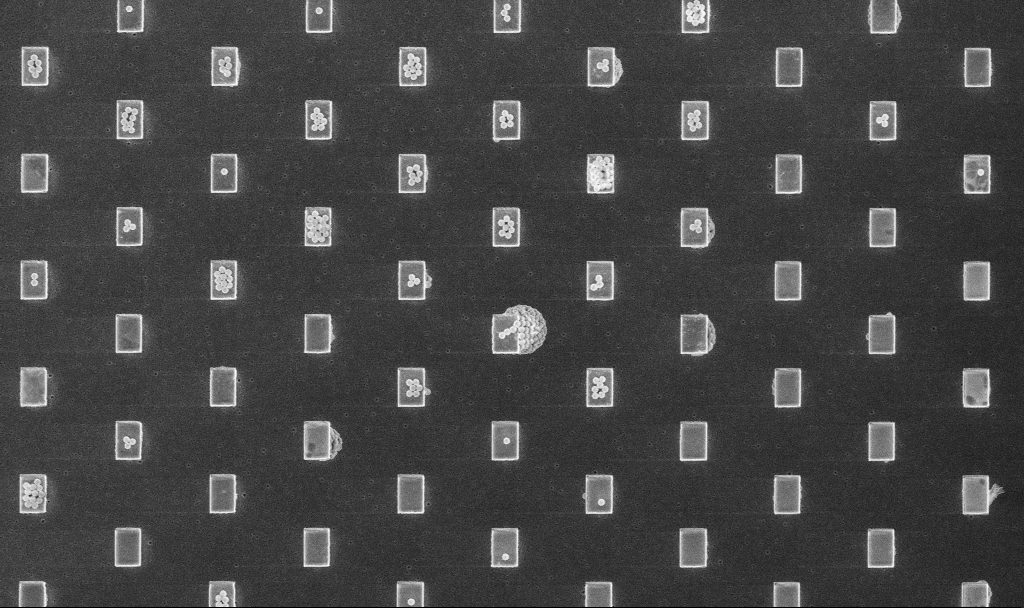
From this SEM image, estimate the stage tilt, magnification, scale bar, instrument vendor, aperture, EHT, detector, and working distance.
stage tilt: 0°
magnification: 3.3 K X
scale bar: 10000 nm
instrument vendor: Zeiss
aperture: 30 µm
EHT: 5 kV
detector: InLens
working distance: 3.2 mm